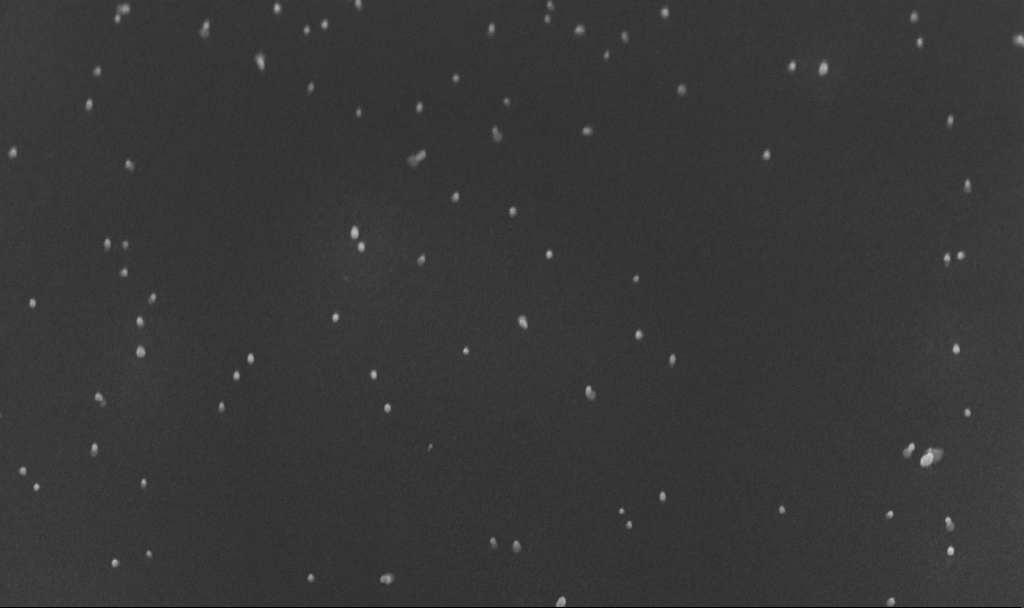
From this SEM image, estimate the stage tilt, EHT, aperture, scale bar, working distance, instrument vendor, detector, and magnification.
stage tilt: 45°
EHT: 10 kV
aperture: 30 µm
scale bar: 200 nm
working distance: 4.8 mm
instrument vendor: Zeiss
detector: InLens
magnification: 100 K X